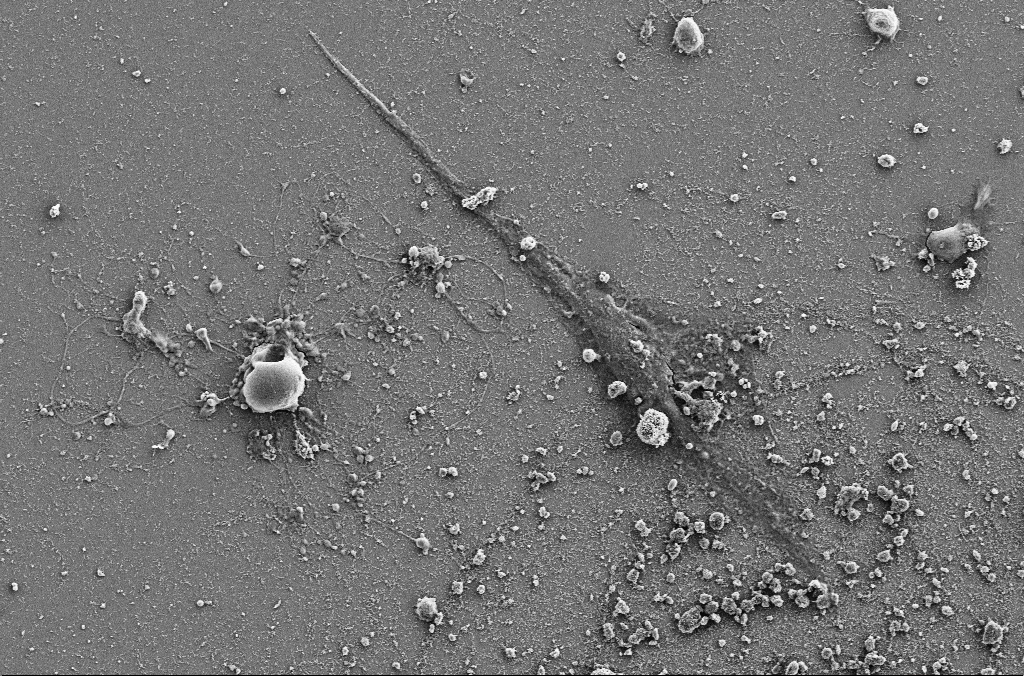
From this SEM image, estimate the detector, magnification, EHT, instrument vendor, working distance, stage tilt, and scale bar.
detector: SE2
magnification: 2 K X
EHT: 5 kV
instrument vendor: Zeiss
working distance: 4 mm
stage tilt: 0°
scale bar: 20000 nm